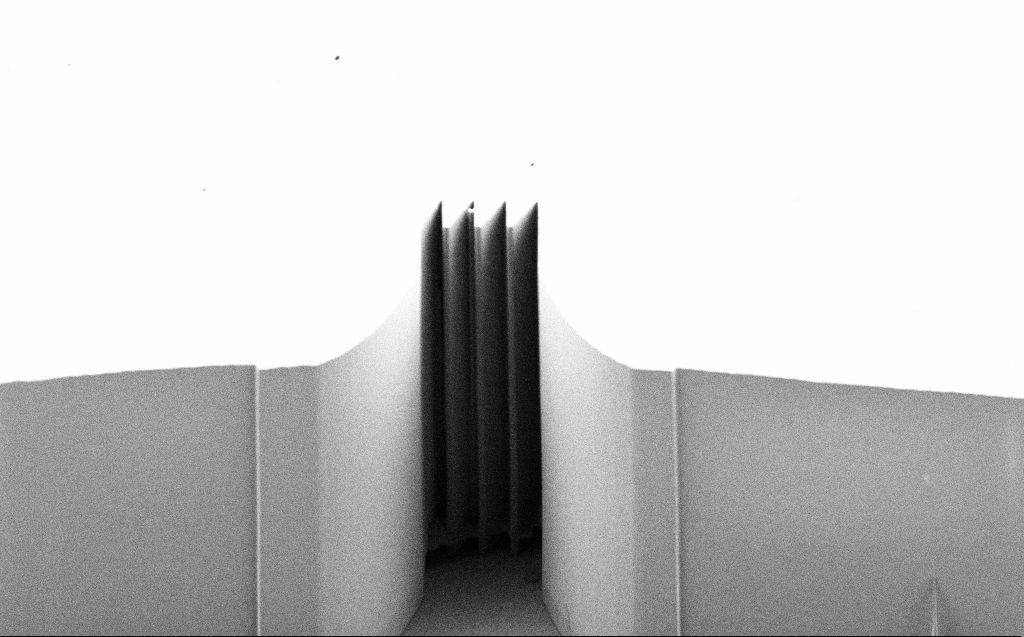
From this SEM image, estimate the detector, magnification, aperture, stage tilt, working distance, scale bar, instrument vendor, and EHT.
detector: SE2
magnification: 1.04 K X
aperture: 30 µm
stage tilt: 45°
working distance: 6 mm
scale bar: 20000 nm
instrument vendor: Zeiss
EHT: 1 kV